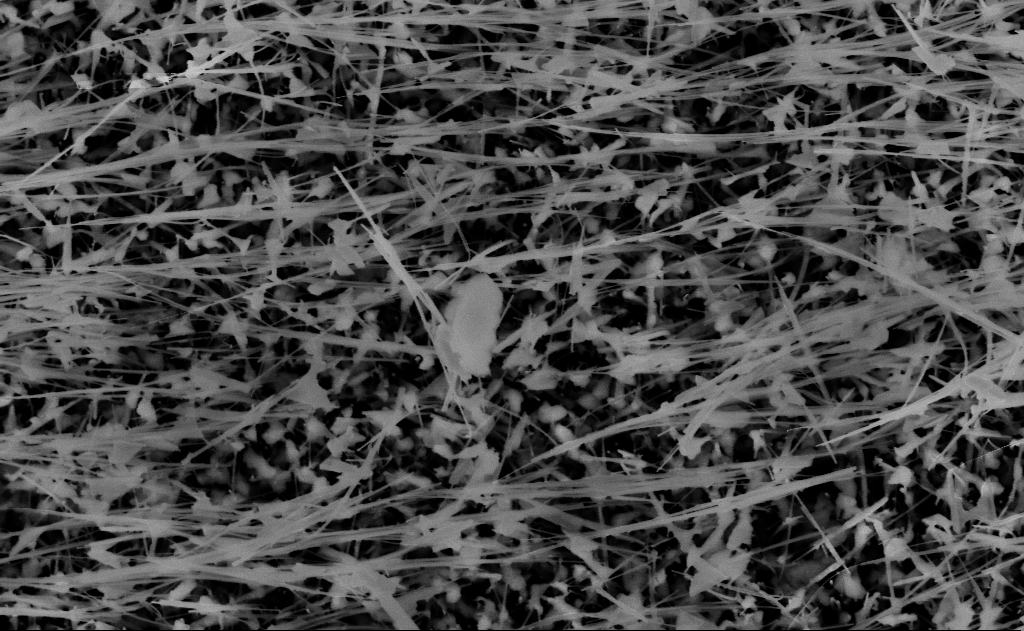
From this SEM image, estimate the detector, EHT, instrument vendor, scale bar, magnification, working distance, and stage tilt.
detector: InLens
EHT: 10 kV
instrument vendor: Zeiss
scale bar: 1000 nm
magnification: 40 K X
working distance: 15 mm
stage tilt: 0°